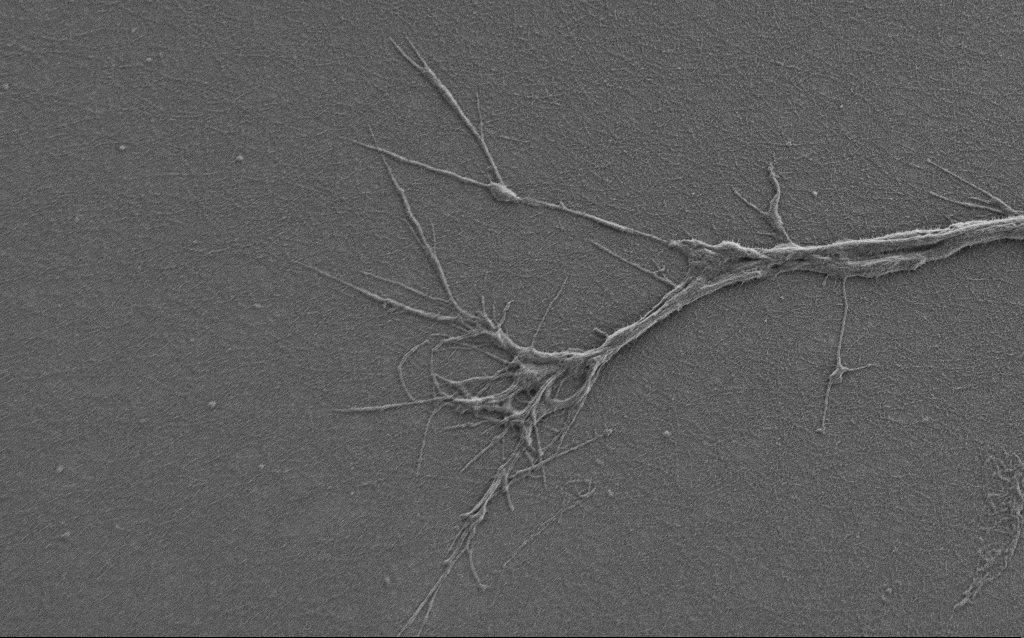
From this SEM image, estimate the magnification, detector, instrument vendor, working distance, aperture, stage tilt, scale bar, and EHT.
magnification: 6 K X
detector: SE2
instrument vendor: Zeiss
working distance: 6 mm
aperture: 30 µm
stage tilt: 0°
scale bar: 10000 nm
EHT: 0.9 kV